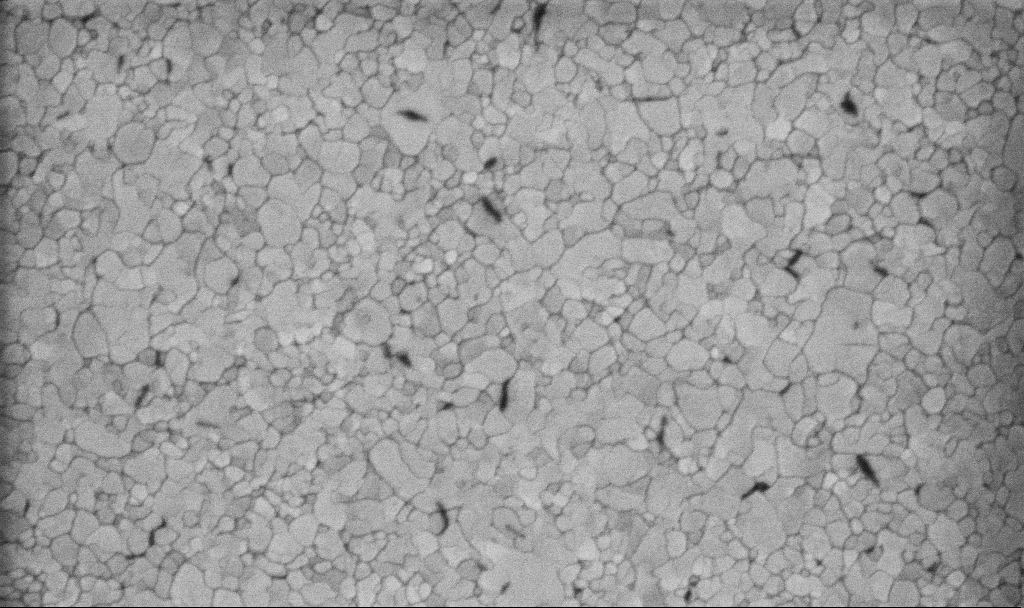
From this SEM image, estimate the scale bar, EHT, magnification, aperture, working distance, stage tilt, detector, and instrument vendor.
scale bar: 200 nm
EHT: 5 kV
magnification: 100.15 K X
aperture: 30 µm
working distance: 3.1 mm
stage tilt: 0°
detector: InLens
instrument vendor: Zeiss